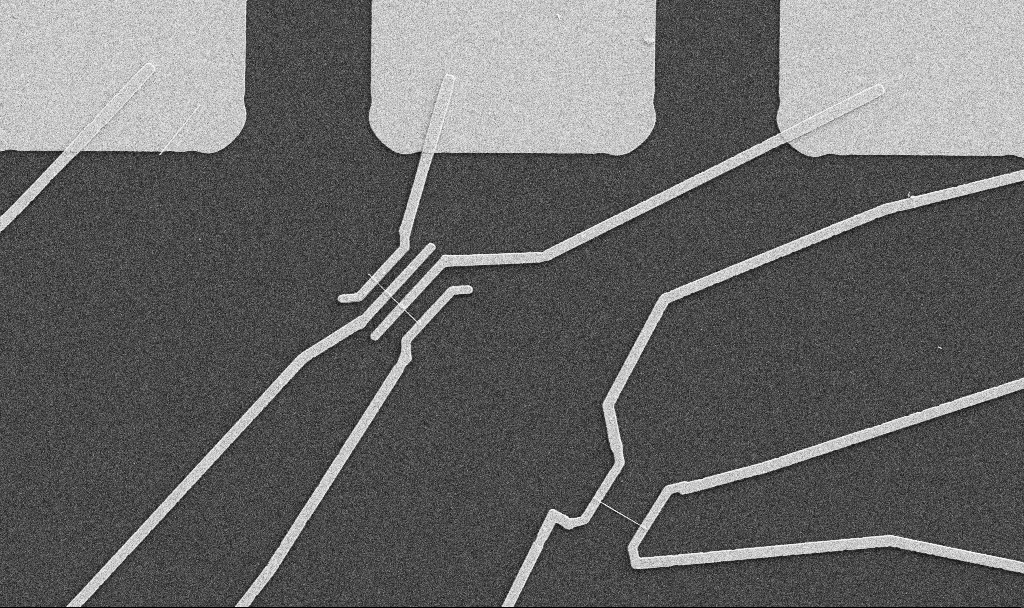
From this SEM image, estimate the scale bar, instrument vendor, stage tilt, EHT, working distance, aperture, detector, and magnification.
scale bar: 10000 nm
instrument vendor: Zeiss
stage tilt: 0°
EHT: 5 kV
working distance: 10.7 mm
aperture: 30 µm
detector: SE2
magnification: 5 K X